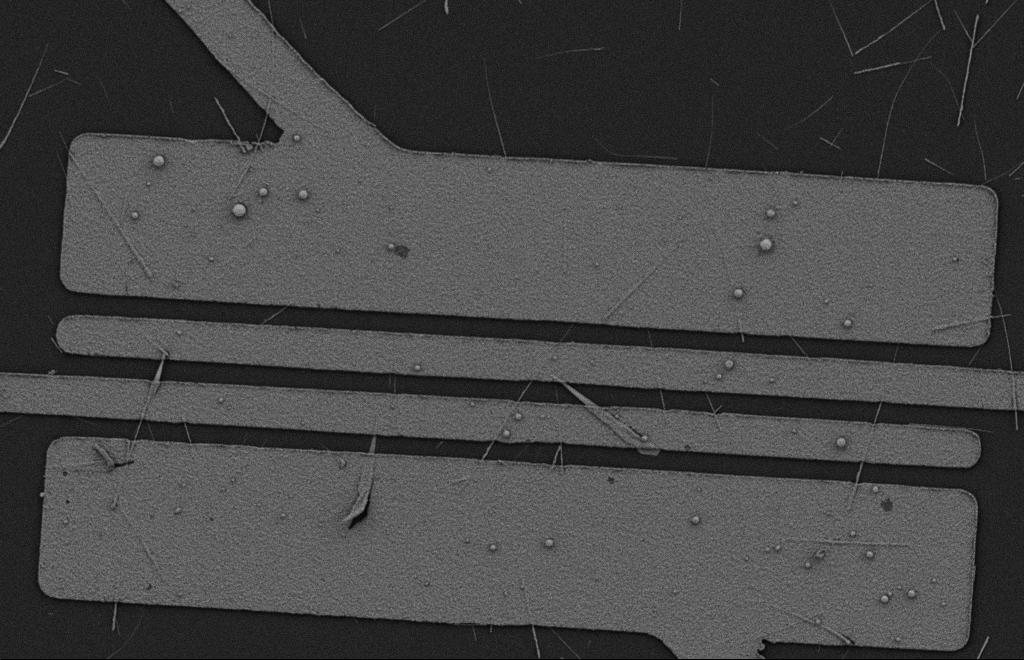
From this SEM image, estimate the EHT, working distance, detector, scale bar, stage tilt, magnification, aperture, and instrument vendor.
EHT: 2 kV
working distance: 10 mm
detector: SE2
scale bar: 2000 nm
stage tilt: -0.3°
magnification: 5.57 K X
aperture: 20 µm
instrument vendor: Zeiss